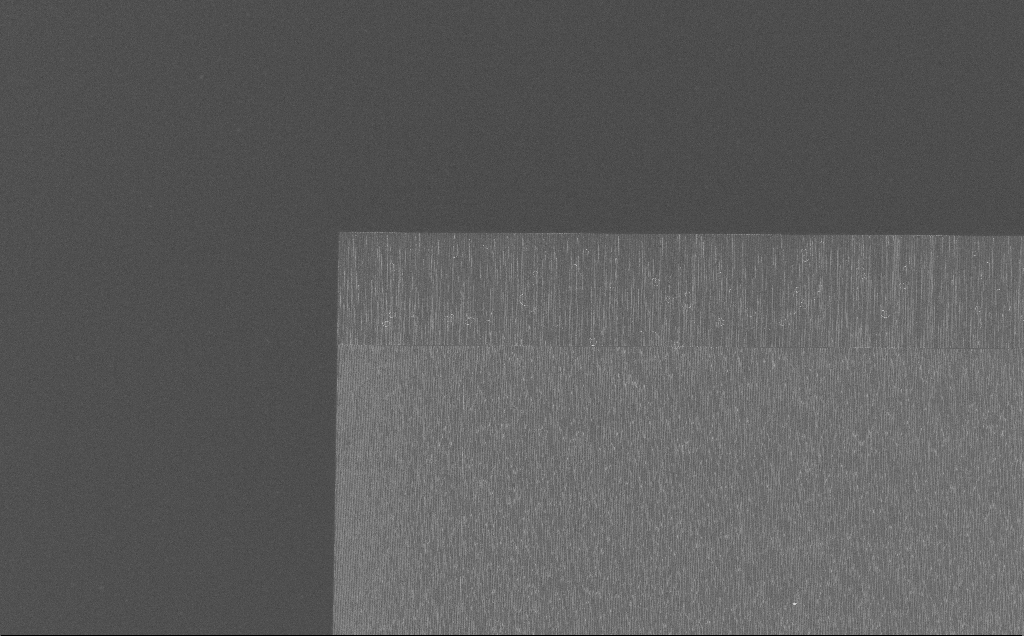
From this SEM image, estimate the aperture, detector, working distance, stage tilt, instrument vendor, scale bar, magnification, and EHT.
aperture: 30 µm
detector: InLens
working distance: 7 mm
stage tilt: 0°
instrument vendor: Zeiss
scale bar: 20000 nm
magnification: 1.05 K X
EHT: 10 kV